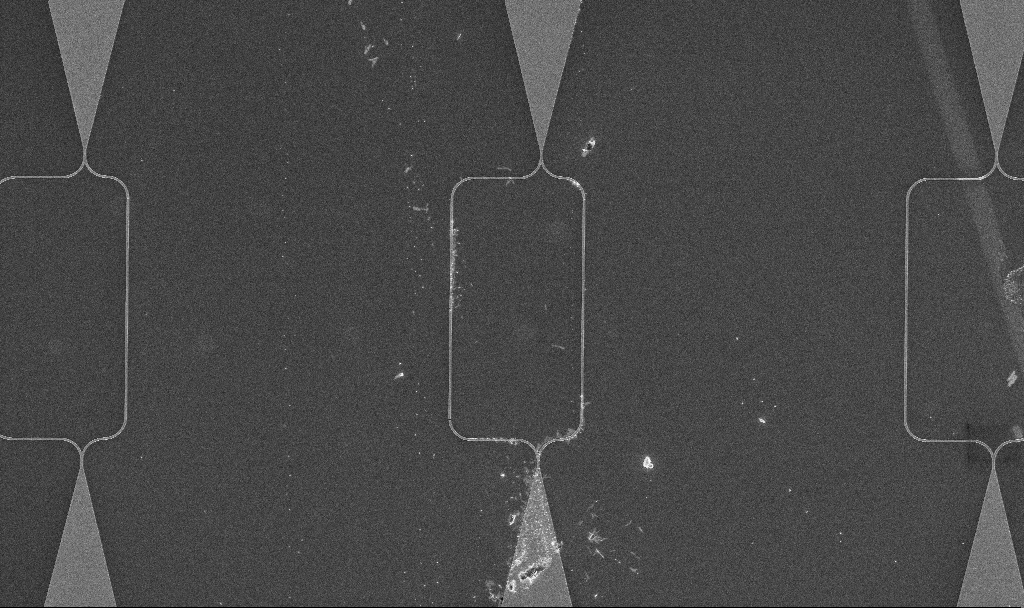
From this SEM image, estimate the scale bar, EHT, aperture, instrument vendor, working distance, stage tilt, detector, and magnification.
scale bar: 10000 nm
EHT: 5 kV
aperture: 30 µm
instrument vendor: Zeiss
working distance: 10.3 mm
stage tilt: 0°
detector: InLens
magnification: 1.68 K X